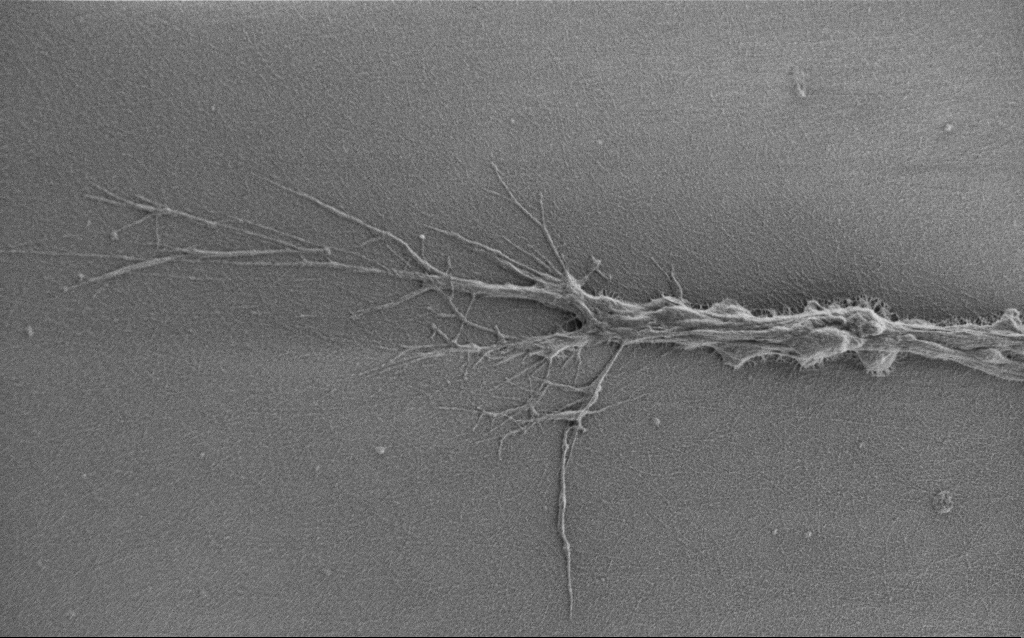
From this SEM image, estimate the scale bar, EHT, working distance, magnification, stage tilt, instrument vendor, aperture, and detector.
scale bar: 10000 nm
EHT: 0.9 kV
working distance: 7 mm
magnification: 6 K X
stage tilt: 0°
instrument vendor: Zeiss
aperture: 30 µm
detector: SE2